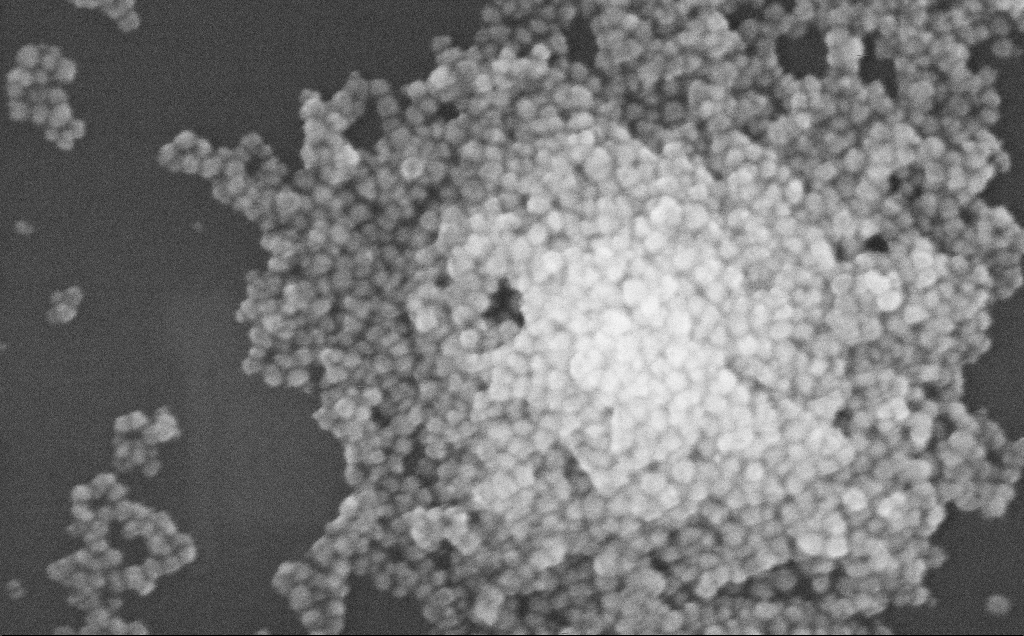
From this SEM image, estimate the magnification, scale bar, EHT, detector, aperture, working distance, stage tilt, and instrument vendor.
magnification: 294.88 K X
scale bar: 200 nm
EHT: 10 kV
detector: InLens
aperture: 30 µm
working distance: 3.7 mm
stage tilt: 0°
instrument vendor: Zeiss